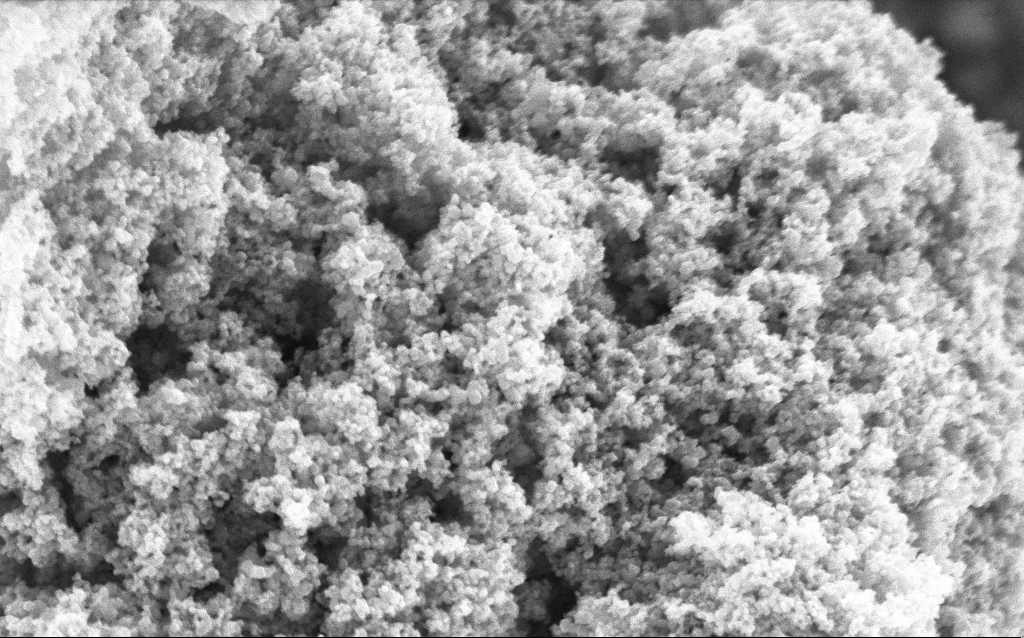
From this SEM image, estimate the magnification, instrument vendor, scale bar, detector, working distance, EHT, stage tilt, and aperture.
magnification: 88.07 K X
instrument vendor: Zeiss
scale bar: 200 nm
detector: InLens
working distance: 4.6 mm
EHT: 5 kV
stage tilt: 0°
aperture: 30 µm